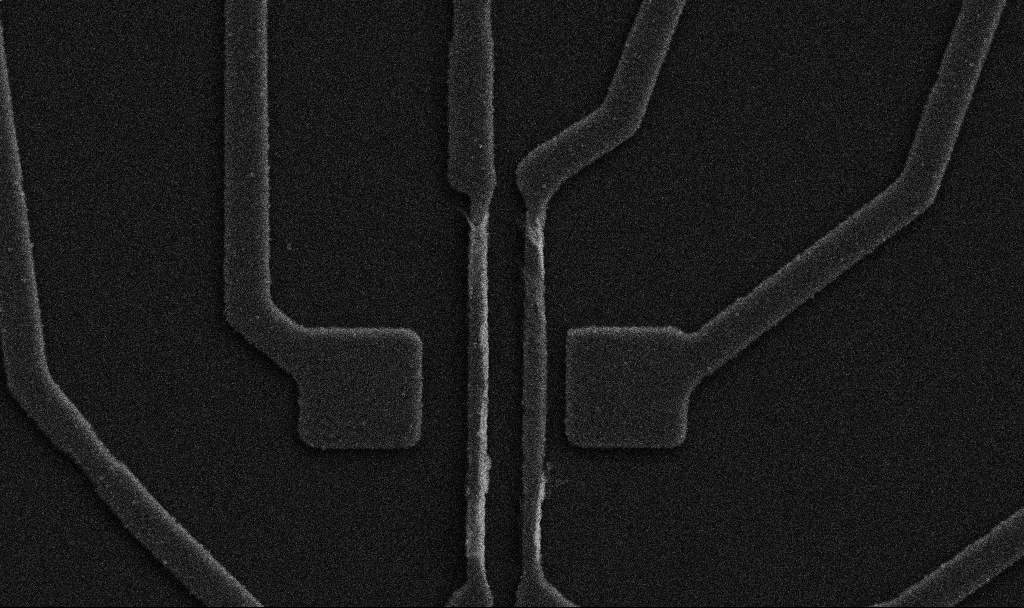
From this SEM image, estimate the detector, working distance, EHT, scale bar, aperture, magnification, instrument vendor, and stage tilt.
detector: SE2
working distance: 10.7 mm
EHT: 5 kV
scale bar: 1000 nm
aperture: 30 µm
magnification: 20 K X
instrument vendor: Zeiss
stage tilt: -0°